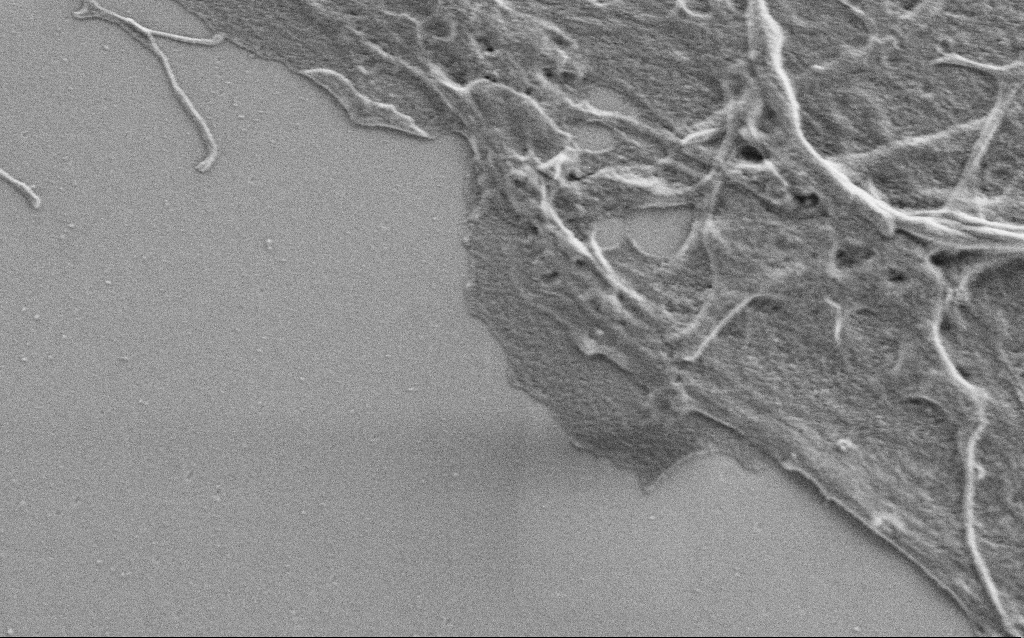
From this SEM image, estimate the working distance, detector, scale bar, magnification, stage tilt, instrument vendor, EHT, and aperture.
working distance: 4 mm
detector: SE2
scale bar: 2000 nm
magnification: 20 K X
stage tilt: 0°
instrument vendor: Zeiss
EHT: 0.9 kV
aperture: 30 µm